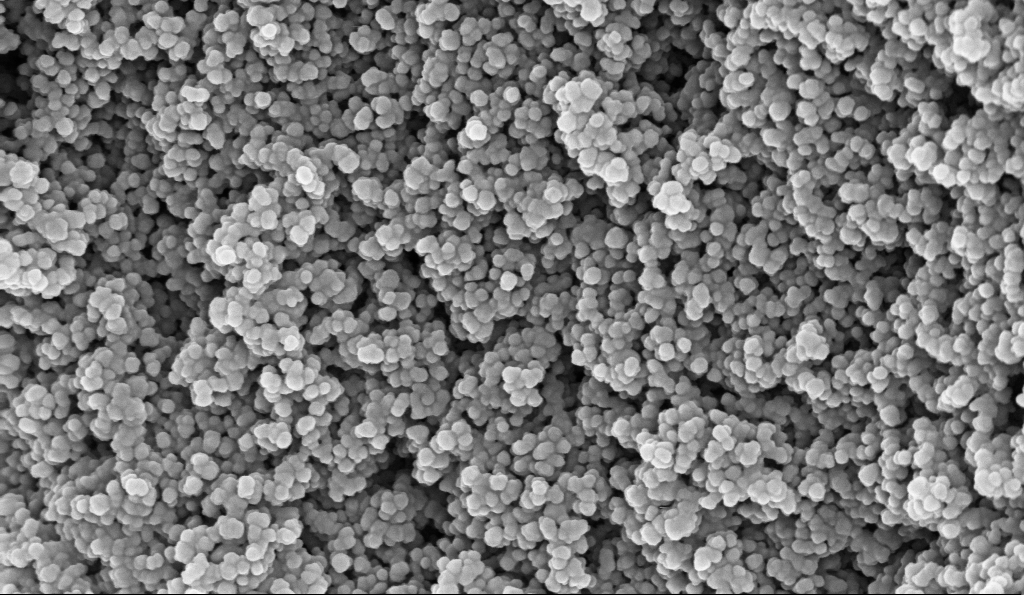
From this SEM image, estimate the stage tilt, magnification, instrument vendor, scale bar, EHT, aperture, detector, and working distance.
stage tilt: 0°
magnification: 135 K X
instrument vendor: Zeiss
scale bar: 100 nm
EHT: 10 kV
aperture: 30 µm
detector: InLens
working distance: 5.1 mm